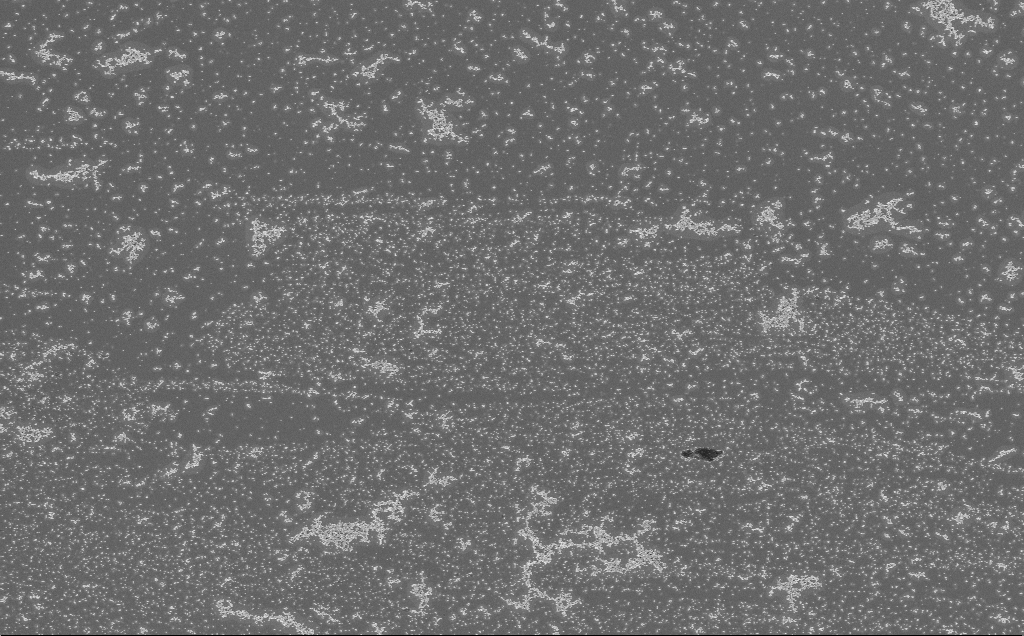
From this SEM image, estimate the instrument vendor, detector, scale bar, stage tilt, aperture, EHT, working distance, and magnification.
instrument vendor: Zeiss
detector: InLens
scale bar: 10000 nm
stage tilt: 0°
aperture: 30 µm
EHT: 3 kV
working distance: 5 mm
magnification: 5 K X